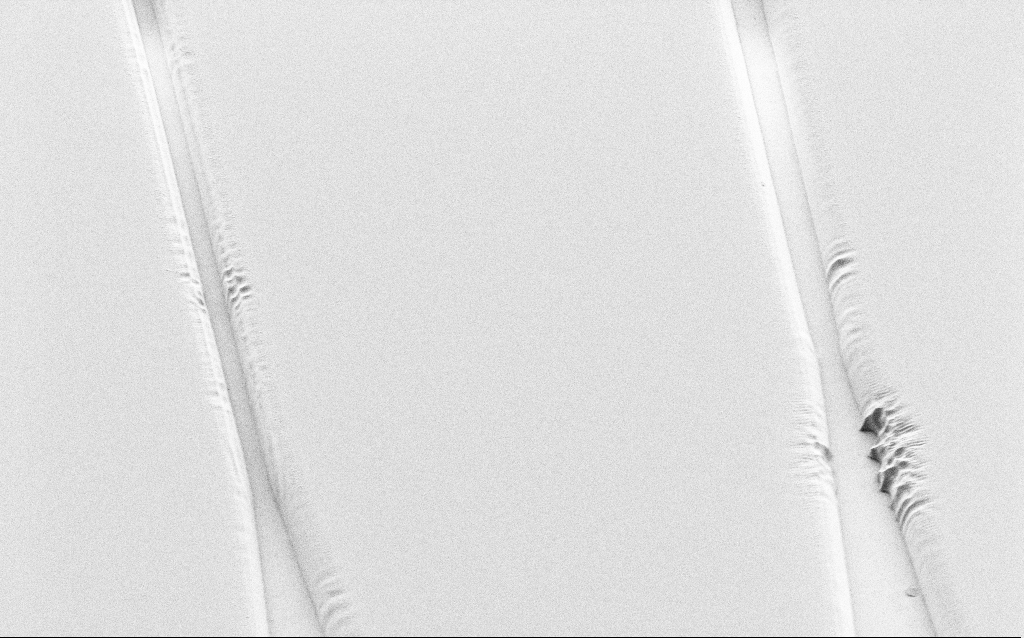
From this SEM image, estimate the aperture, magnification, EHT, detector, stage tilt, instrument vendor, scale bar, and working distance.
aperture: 30 µm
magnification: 2.08 K X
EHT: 1 kV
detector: SE2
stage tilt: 36°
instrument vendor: Zeiss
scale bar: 20000 nm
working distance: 6 mm